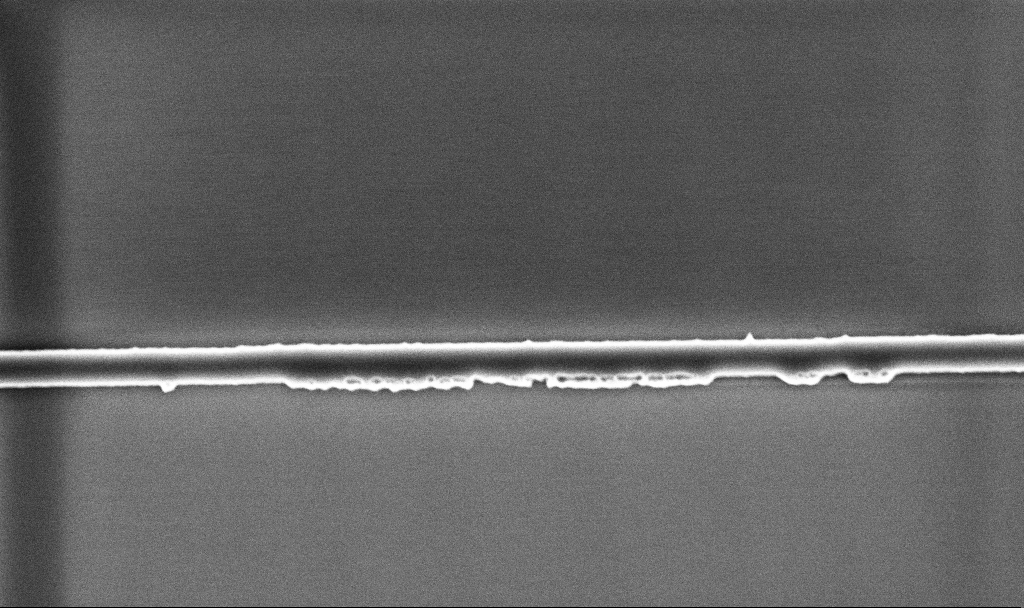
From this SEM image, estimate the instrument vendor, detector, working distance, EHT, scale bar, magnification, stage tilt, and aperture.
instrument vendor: Zeiss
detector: InLens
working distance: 5.2 mm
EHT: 5 kV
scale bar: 2000 nm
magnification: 26.25 K X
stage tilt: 0°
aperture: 30 µm